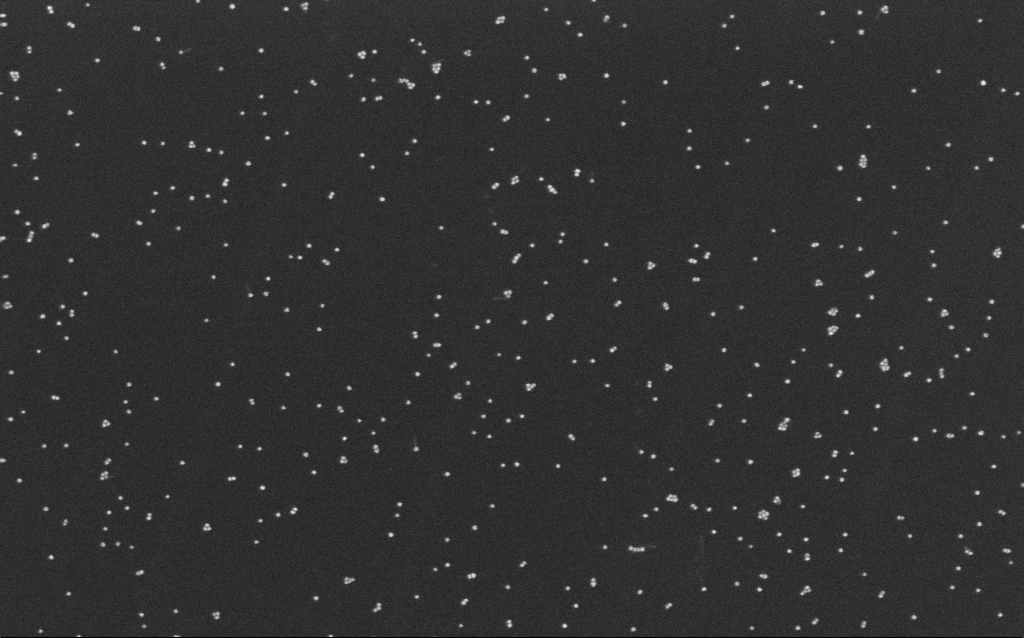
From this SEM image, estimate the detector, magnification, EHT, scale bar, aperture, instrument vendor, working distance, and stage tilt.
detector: InLens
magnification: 100 K X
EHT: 10 kV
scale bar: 200 nm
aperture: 30 µm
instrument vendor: Zeiss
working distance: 6.5 mm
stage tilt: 0°